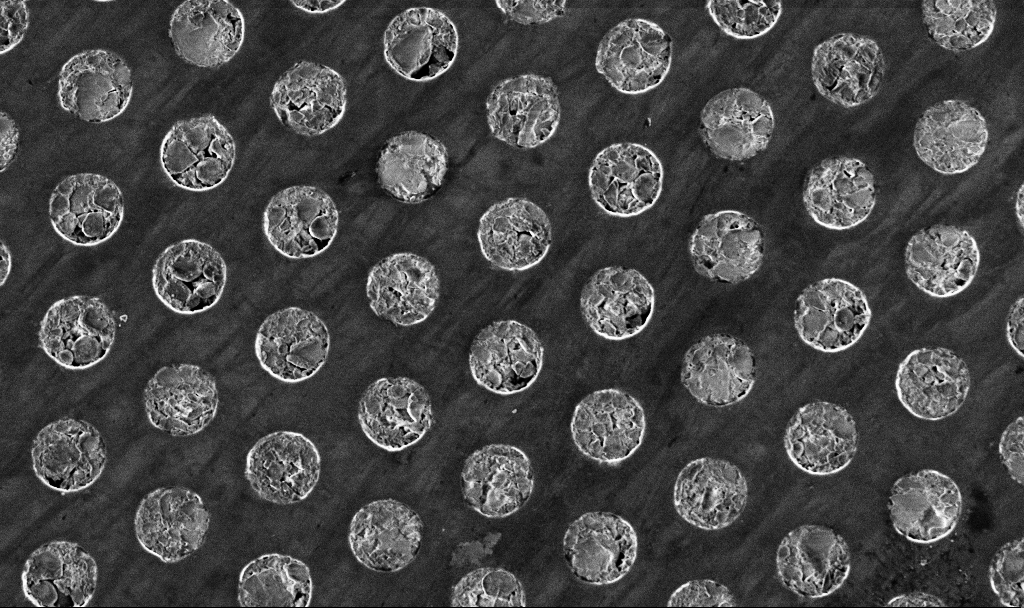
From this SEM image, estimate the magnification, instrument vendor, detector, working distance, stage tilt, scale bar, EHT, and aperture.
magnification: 7.57 K X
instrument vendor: Zeiss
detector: InLens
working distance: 5 mm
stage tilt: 0°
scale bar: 2000 nm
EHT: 3 kV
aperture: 30 µm